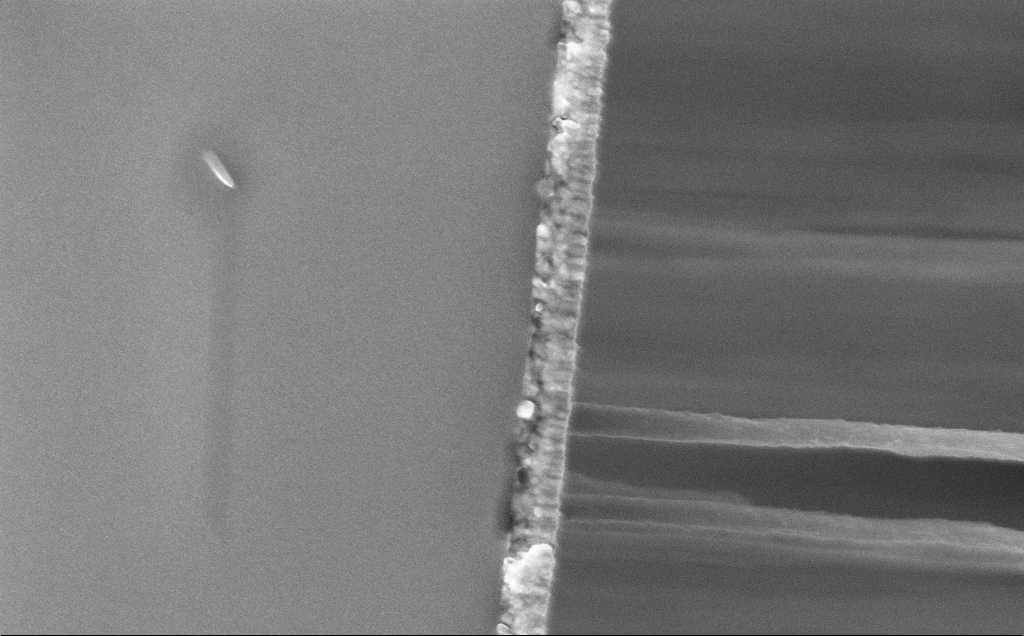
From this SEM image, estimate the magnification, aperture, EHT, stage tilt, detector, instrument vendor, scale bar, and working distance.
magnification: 74.28 K X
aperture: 30 µm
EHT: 5 kV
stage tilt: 0°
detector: InLens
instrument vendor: Zeiss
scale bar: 200 nm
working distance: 12 mm